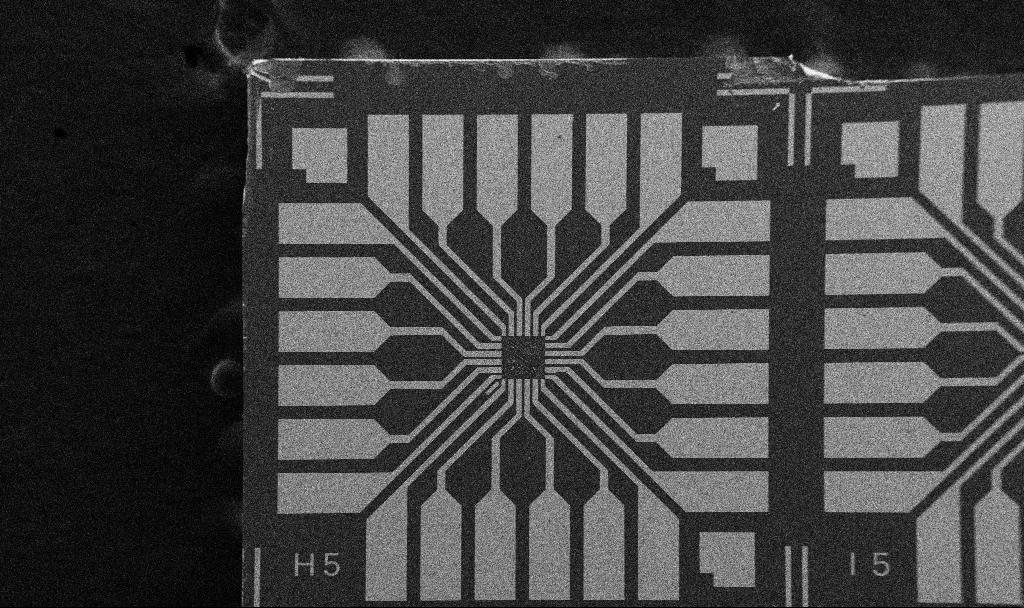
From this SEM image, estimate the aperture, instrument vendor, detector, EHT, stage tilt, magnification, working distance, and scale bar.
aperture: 30 µm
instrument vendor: Zeiss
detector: SE2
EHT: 5 kV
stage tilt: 0°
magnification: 0.1 K X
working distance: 10.7 mm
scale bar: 200000 nm